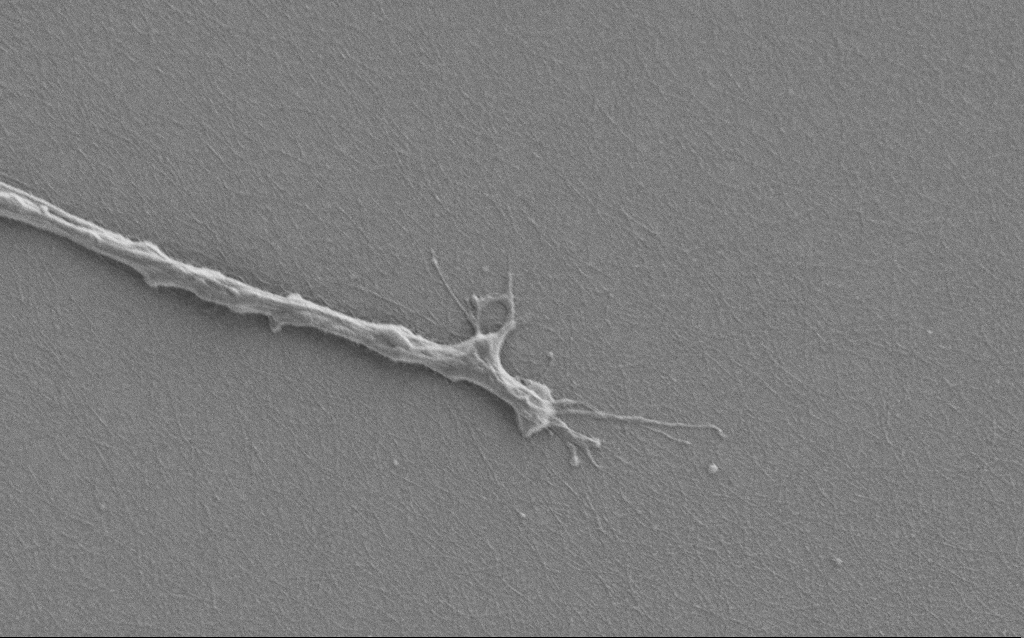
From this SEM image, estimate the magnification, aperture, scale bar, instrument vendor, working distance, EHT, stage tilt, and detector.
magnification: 7.5 K X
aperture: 30 µm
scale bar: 2000 nm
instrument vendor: Zeiss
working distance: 6 mm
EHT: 1 kV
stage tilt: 0°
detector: SE2